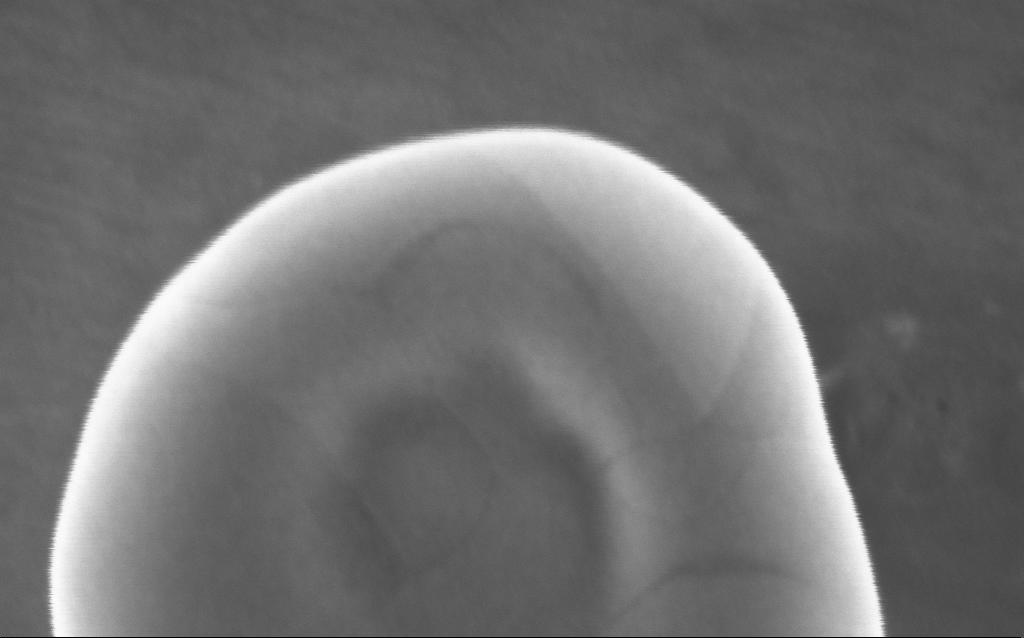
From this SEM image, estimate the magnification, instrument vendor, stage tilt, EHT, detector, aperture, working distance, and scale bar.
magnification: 451 K X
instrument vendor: Zeiss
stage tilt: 0°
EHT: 5 kV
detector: InLens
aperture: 30 µm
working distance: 2 mm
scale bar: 100 nm